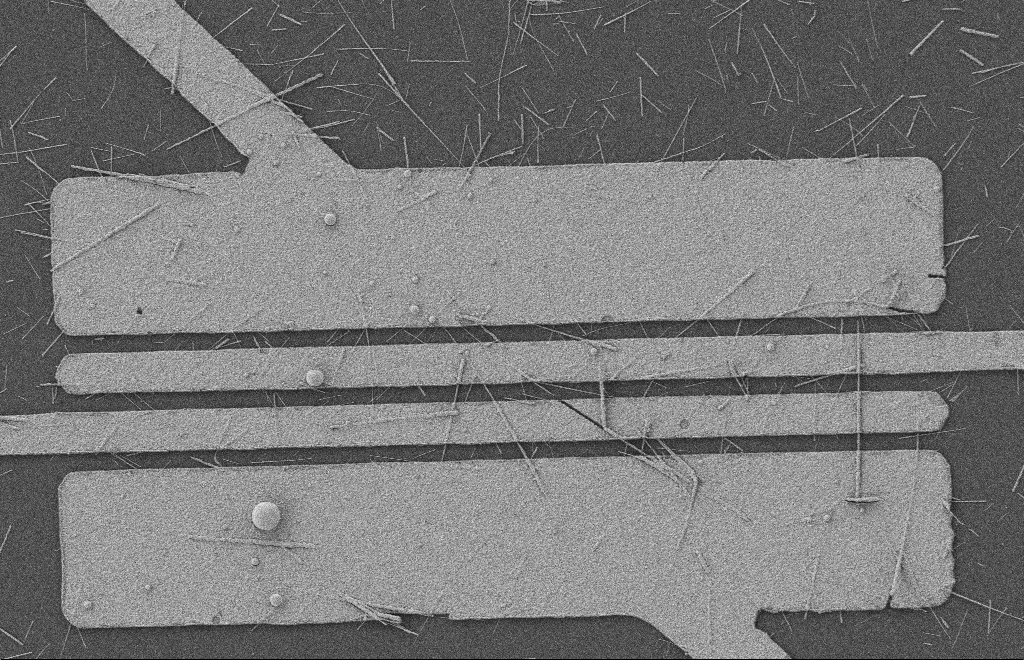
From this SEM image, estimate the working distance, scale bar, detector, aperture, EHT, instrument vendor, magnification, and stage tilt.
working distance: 8 mm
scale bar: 2000 nm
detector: SE2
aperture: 20 µm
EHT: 2 kV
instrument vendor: Zeiss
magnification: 5.37 K X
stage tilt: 0°